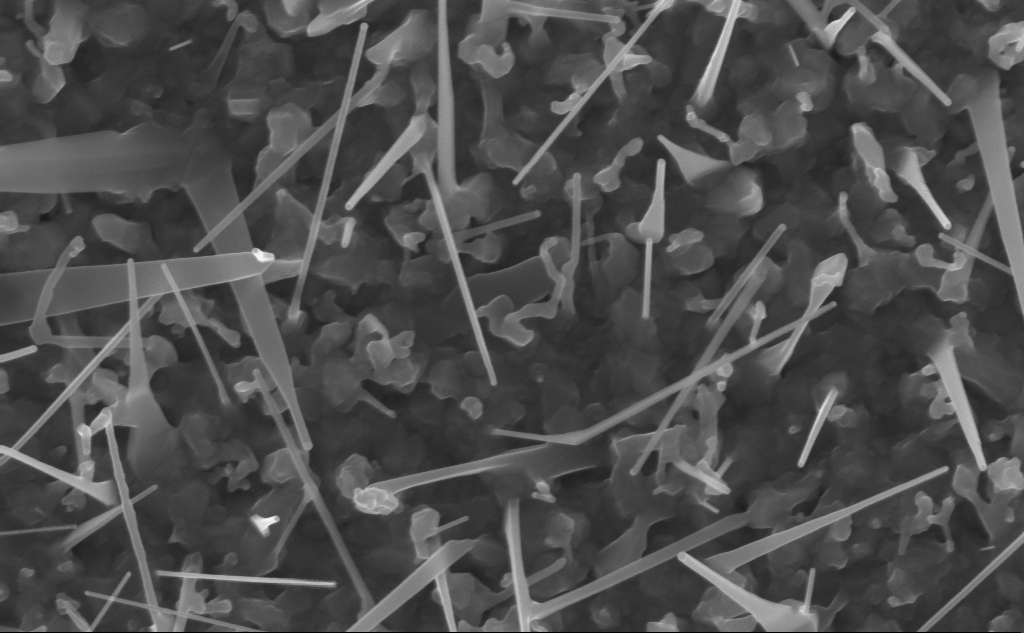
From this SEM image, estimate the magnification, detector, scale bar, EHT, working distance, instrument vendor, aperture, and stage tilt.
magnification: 80 K X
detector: InLens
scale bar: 200 nm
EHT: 10 kV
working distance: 5 mm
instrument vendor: Zeiss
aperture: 30 µm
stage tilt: -0°